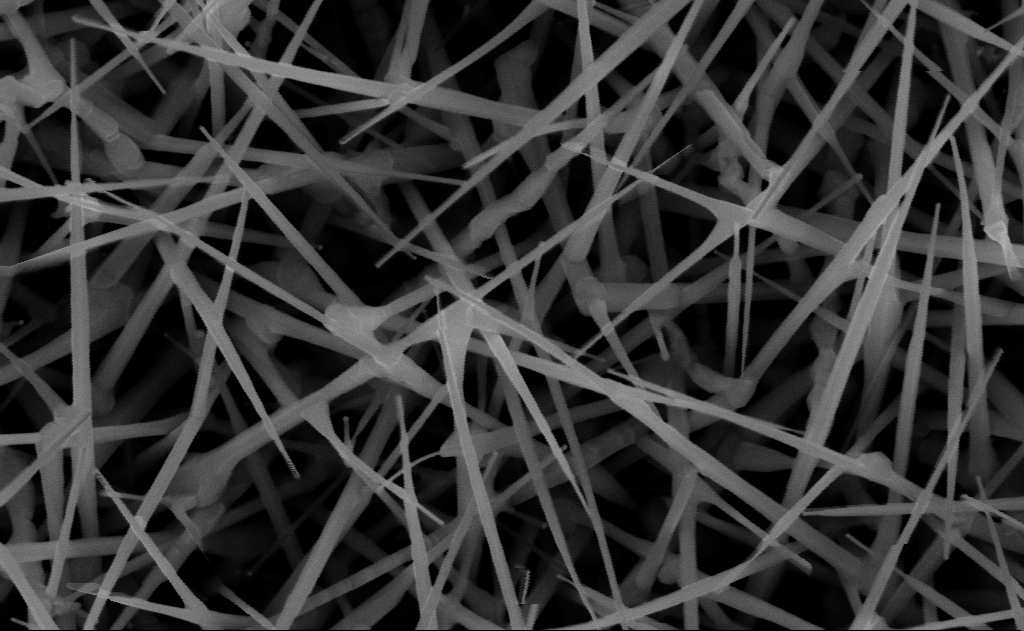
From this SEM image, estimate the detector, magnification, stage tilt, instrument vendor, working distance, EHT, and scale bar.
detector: InLens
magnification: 80 K X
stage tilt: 0°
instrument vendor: Zeiss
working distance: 15 mm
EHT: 10 kV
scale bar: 200 nm